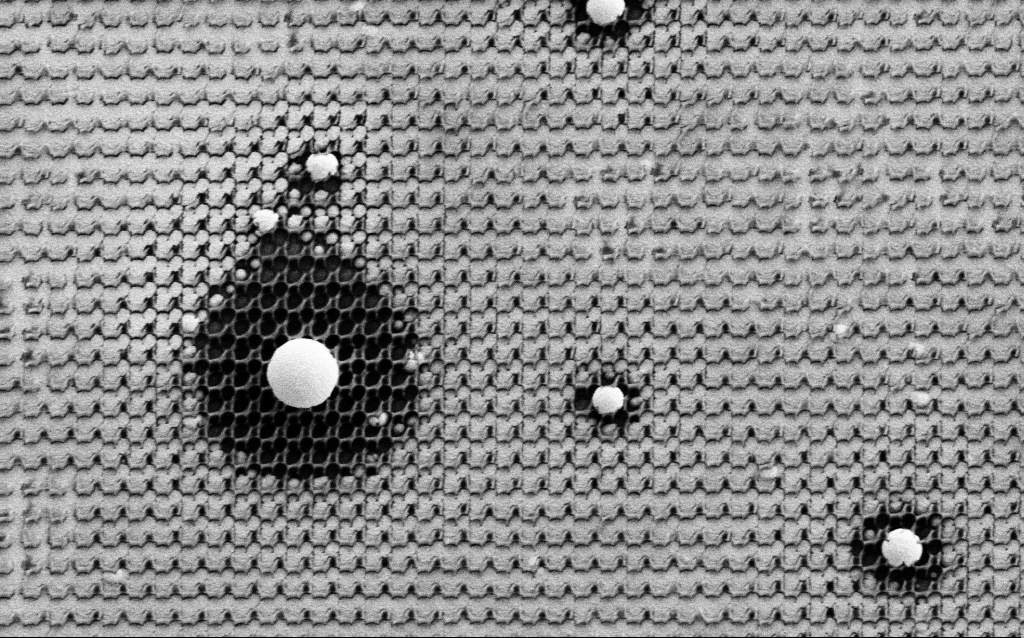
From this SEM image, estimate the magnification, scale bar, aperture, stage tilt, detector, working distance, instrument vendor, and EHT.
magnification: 20.61 K X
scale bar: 2000 nm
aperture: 30 µm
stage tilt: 0°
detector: SE2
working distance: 8 mm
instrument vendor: Zeiss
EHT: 1.5 kV